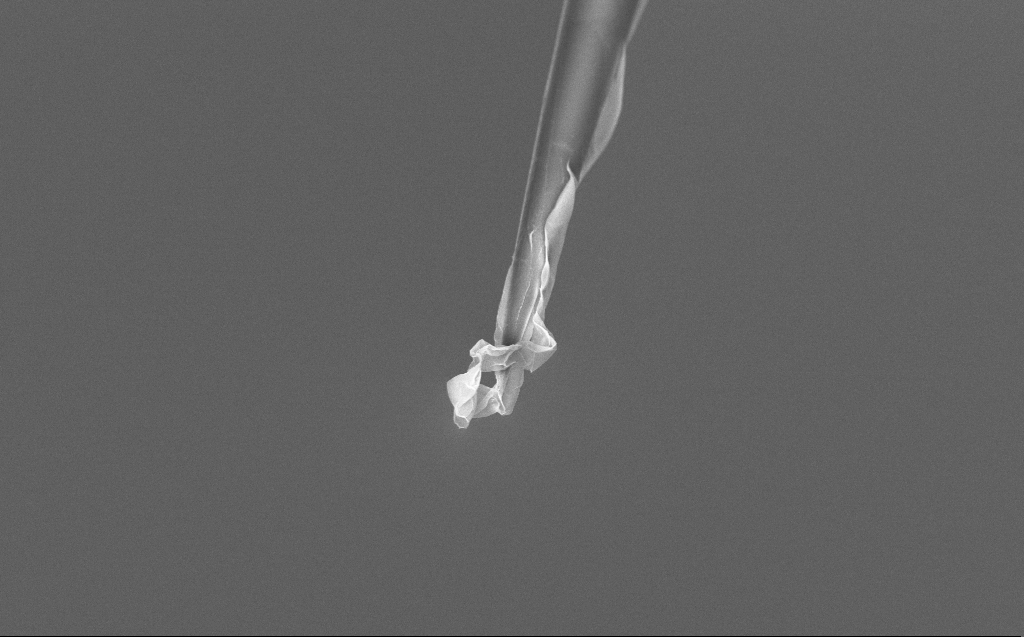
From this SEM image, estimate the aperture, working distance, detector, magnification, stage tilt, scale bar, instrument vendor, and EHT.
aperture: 30 µm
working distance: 6 mm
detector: InLens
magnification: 10 K X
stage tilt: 45°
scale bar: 2000 nm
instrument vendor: Zeiss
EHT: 5 kV